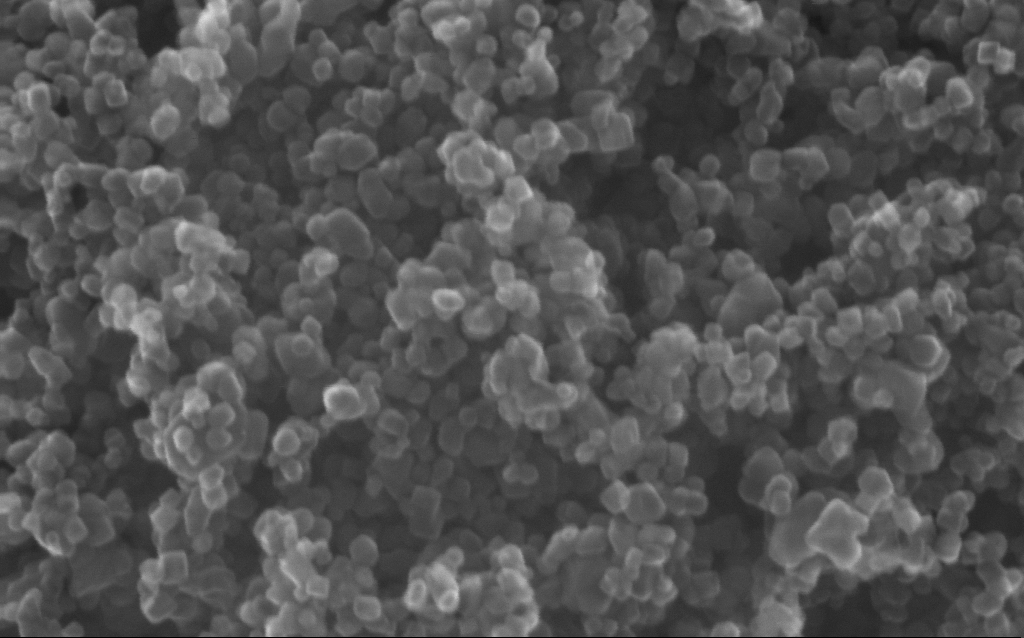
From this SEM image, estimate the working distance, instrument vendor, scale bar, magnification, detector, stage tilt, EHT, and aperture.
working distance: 3 mm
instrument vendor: Zeiss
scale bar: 100 nm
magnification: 416 K X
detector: InLens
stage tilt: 0°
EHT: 20 kV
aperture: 30 µm